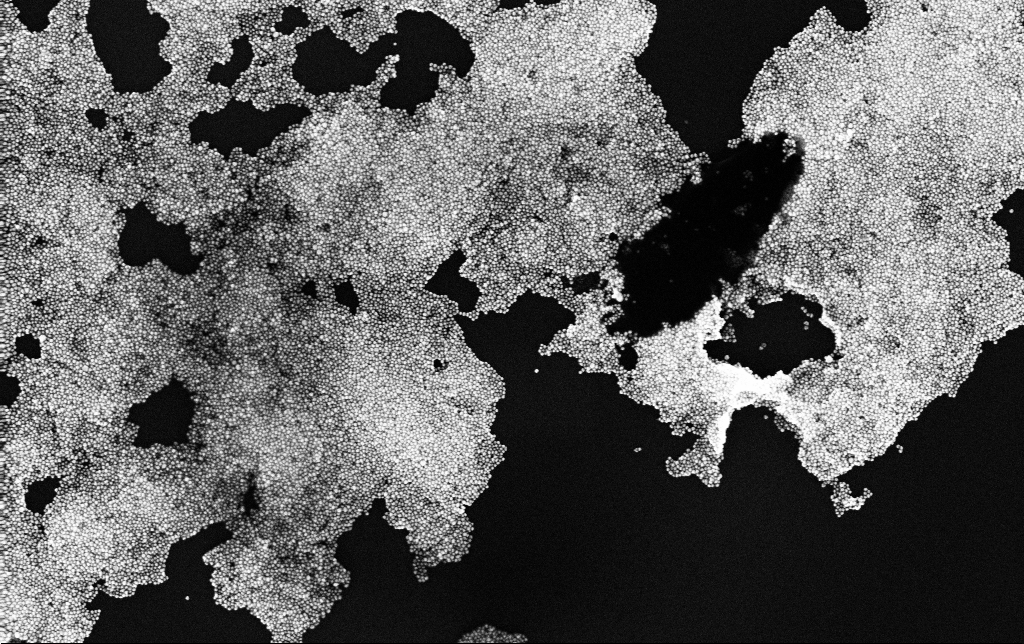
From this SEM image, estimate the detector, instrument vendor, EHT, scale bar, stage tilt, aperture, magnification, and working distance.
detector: InLens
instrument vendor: Zeiss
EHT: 10 kV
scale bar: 200 nm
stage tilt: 0°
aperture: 30 µm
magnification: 100 K X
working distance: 3.4 mm